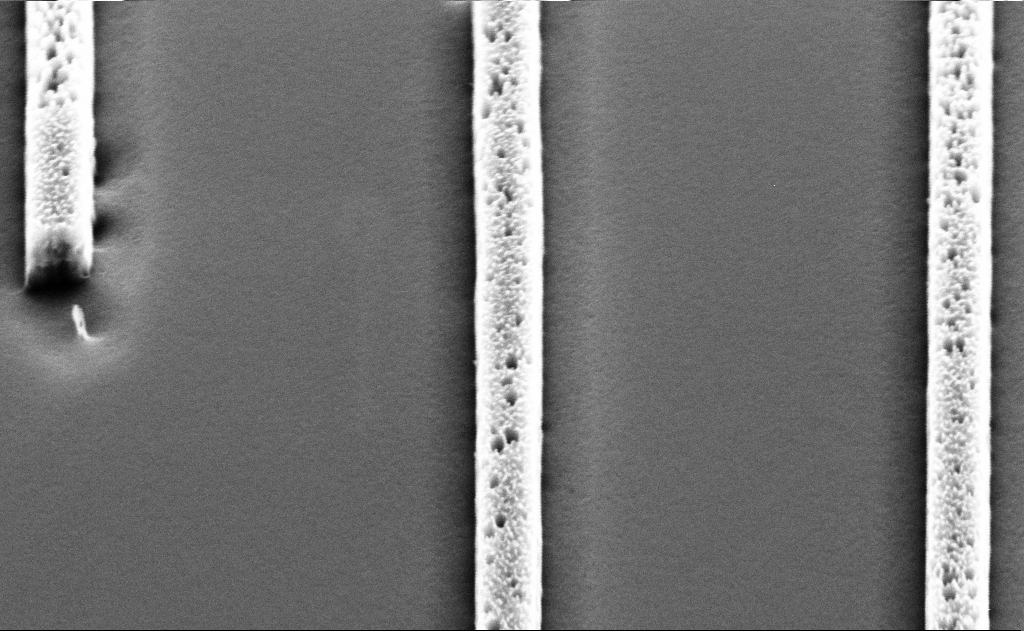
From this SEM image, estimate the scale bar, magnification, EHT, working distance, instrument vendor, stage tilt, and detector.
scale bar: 1000 nm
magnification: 41.19 K X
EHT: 5 kV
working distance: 11 mm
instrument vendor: Zeiss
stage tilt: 45°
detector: SE2